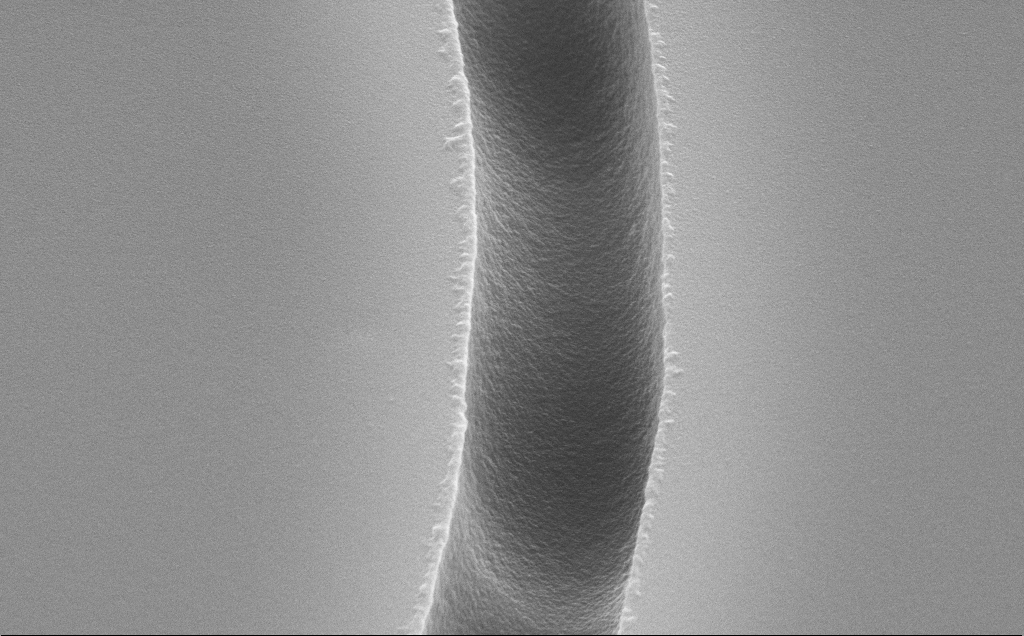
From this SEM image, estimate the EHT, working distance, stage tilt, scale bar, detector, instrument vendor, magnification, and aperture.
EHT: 10 kV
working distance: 11 mm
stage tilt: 45°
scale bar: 1000 nm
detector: SE2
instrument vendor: Zeiss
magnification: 53.53 K X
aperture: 30 µm